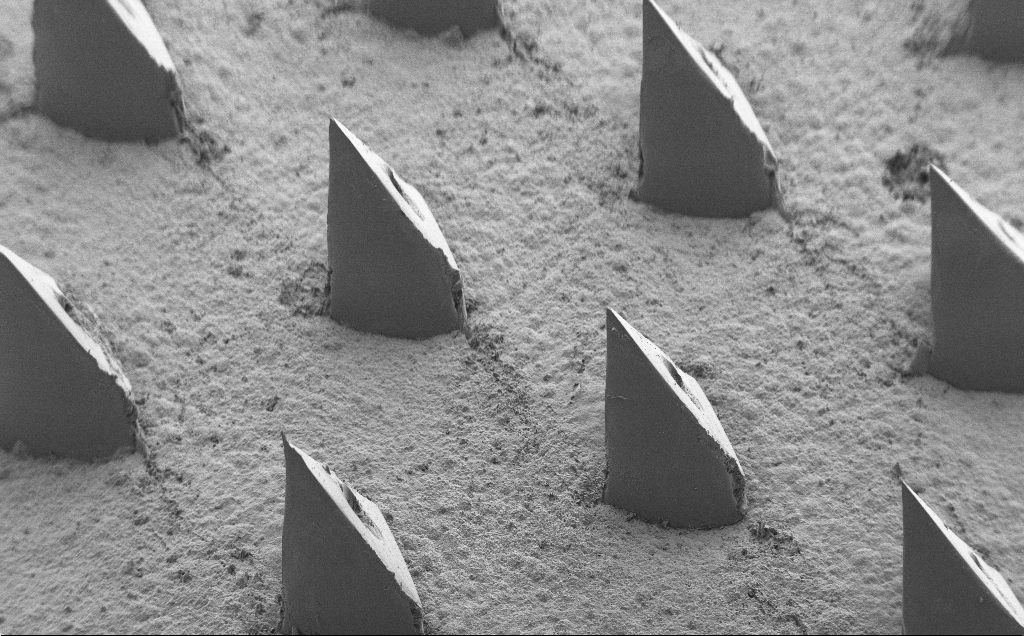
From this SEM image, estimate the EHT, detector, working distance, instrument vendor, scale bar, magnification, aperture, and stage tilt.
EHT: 5 kV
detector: SE2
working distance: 9 mm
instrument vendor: Zeiss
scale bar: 200000 nm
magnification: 0.087 K X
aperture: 30 µm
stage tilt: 40°